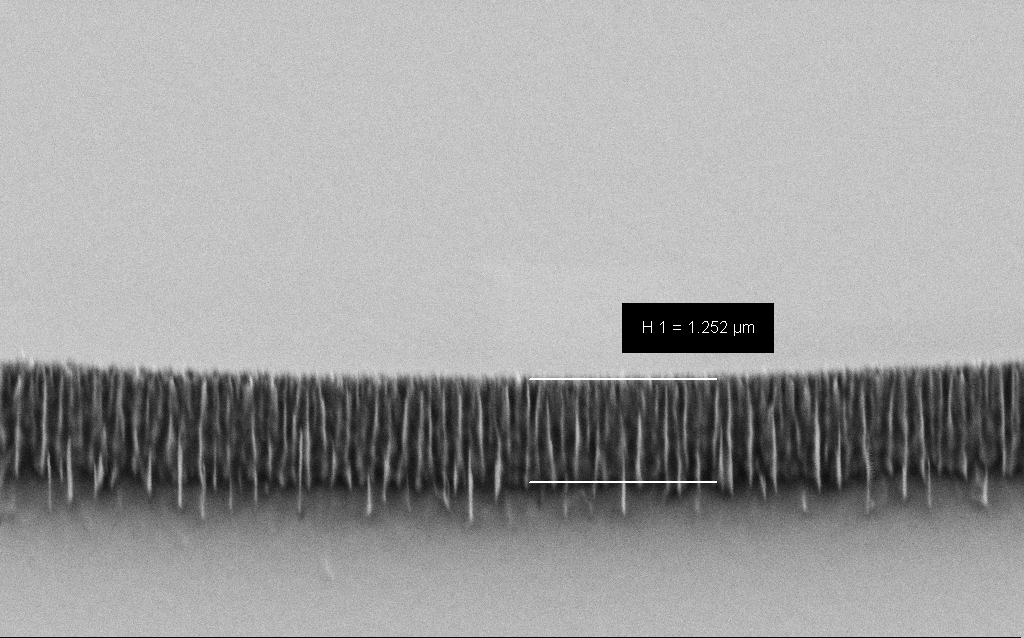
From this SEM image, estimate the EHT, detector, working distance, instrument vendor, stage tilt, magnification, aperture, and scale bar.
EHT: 3 kV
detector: SE2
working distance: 6 mm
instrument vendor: Zeiss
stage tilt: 45°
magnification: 30.21 K X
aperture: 30 µm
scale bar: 2000 nm